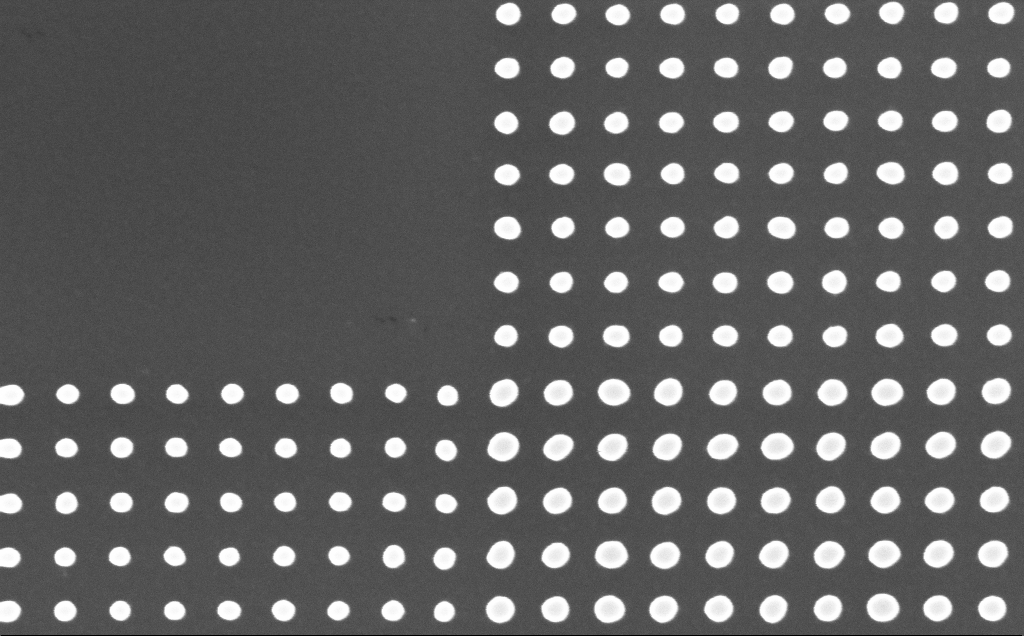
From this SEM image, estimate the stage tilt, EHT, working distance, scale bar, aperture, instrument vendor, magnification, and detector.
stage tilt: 0°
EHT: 10 kV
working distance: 6 mm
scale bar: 200 nm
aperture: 30 µm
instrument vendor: Zeiss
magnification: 100.31 K X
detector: InLens